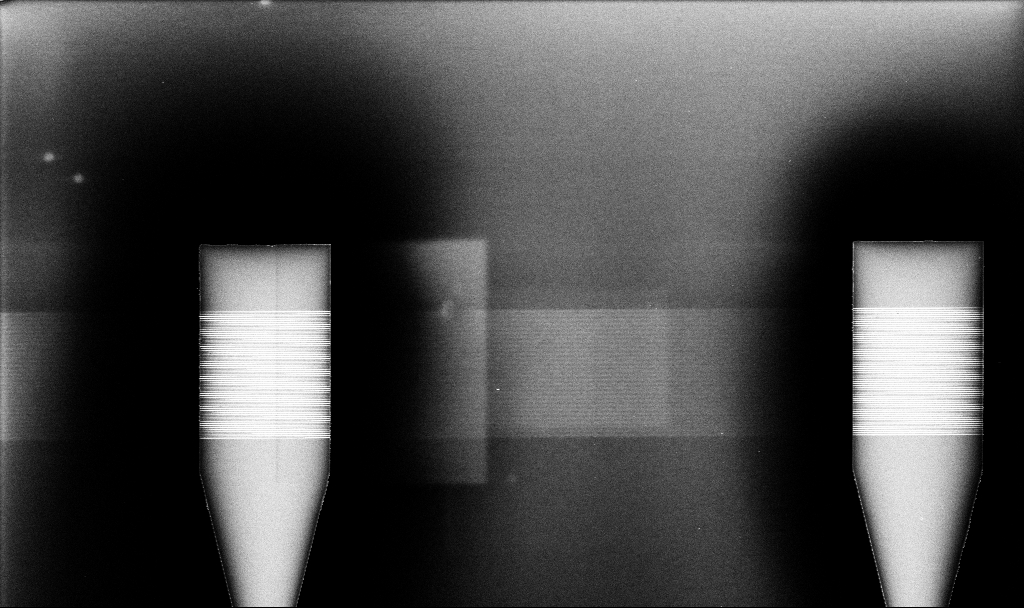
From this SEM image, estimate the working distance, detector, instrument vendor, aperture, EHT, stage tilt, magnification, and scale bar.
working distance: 7.5 mm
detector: InLens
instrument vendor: Zeiss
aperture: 30 µm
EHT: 5 kV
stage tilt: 0°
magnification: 2.4 K X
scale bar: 20000 nm